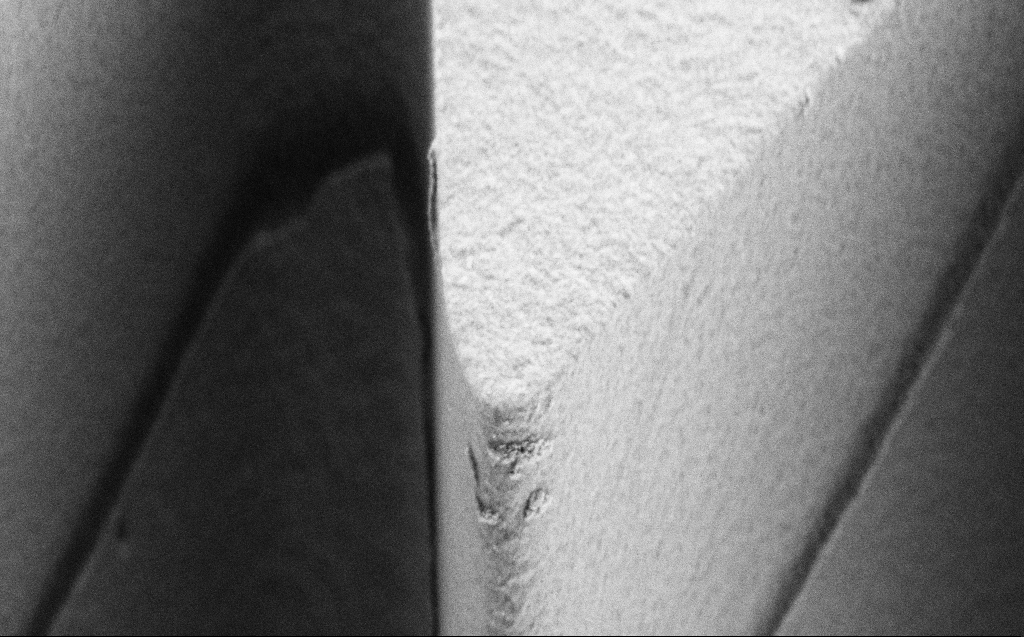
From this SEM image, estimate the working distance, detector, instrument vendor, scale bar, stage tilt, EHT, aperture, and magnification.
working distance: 4 mm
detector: SE2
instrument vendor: Zeiss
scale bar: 1000 nm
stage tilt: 45°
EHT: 3 kV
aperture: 30 µm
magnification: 28.86 K X